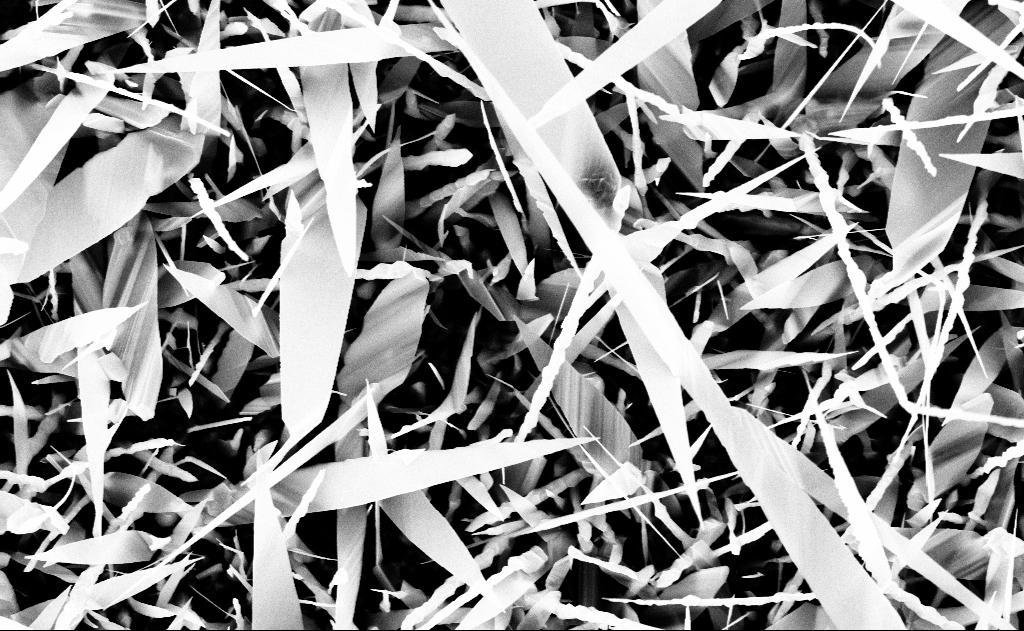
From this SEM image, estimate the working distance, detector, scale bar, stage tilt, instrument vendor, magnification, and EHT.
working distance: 13 mm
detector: InLens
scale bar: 1000 nm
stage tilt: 0°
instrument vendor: Zeiss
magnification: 20 K X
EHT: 10 kV